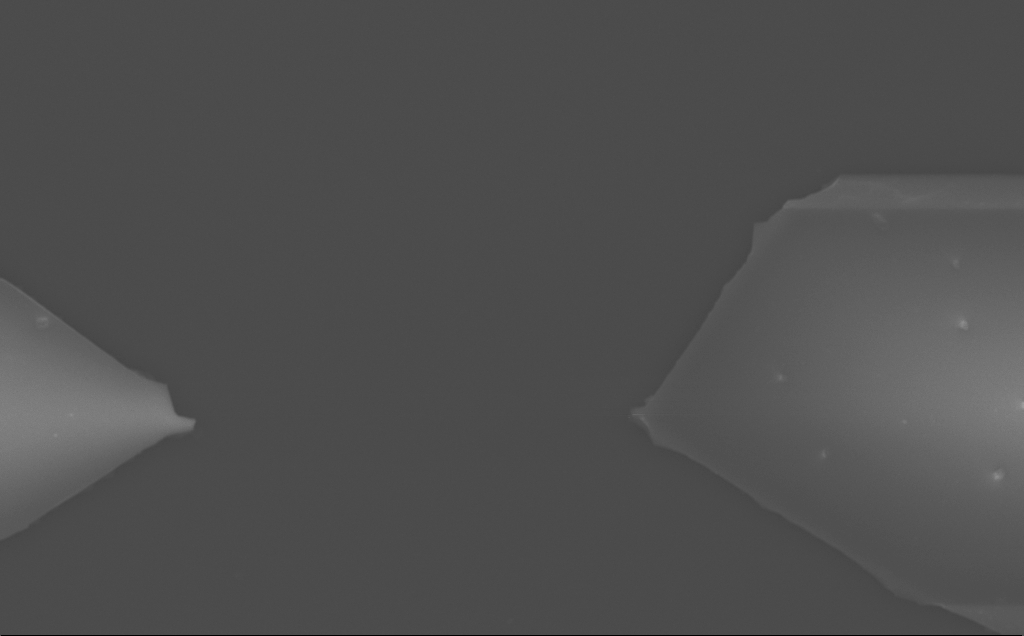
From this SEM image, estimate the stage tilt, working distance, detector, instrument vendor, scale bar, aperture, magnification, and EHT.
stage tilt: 0°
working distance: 10 mm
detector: InLens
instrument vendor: Zeiss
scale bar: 2000 nm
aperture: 30 µm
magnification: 7.64 K X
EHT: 10 kV